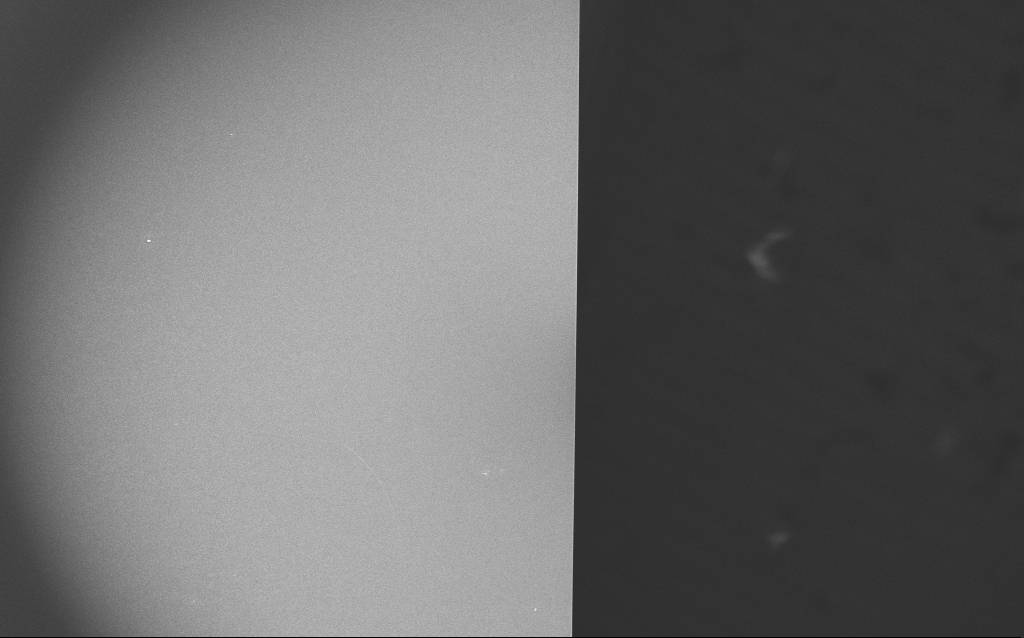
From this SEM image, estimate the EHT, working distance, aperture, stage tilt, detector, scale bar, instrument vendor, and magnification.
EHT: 20 kV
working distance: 5.5 mm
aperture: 30 µm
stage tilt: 0°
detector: InLens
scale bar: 200000 nm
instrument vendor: Zeiss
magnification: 0.2 K X